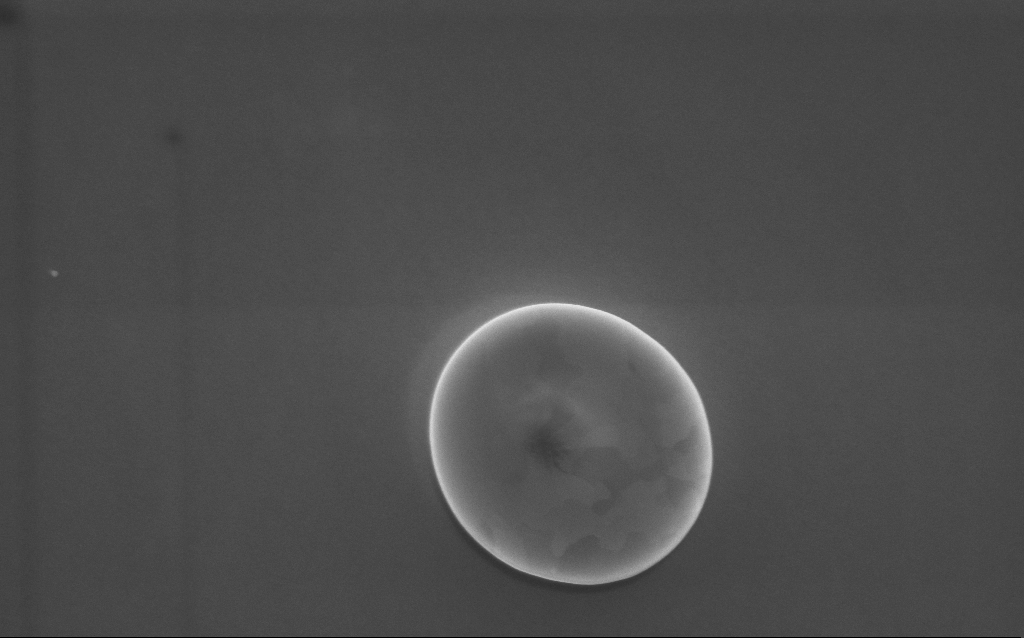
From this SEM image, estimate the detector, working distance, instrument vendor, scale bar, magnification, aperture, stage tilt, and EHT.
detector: InLens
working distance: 3 mm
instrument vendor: Zeiss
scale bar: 1000 nm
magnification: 62 K X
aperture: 30 µm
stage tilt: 0°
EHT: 5 kV